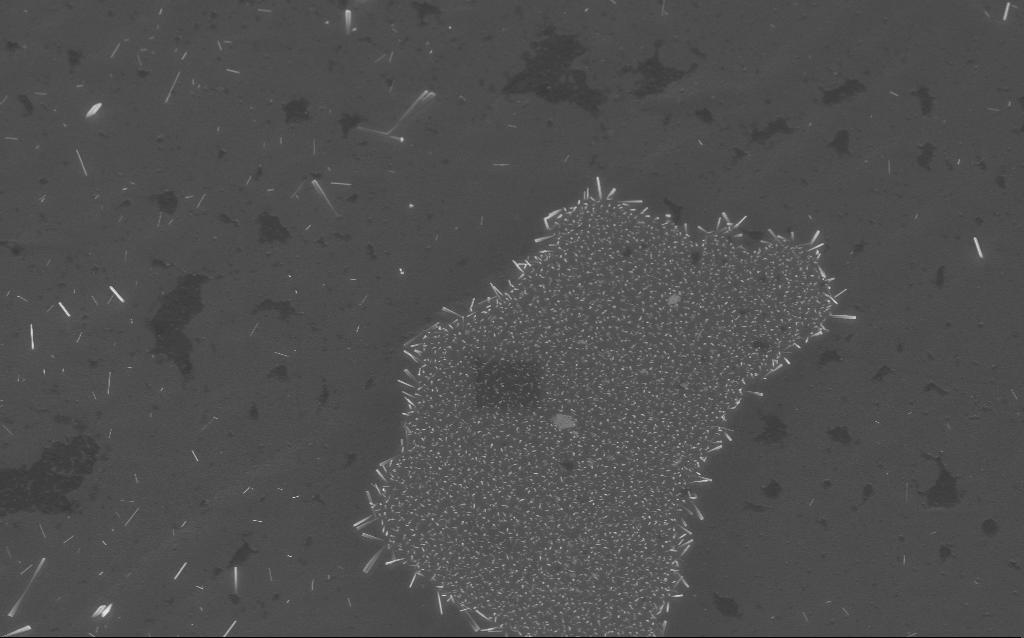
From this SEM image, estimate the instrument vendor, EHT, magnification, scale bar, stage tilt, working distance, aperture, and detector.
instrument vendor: Zeiss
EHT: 5 kV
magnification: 19.83 K X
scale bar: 1000 nm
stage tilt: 23.6°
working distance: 3 mm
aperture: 30 µm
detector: InLens